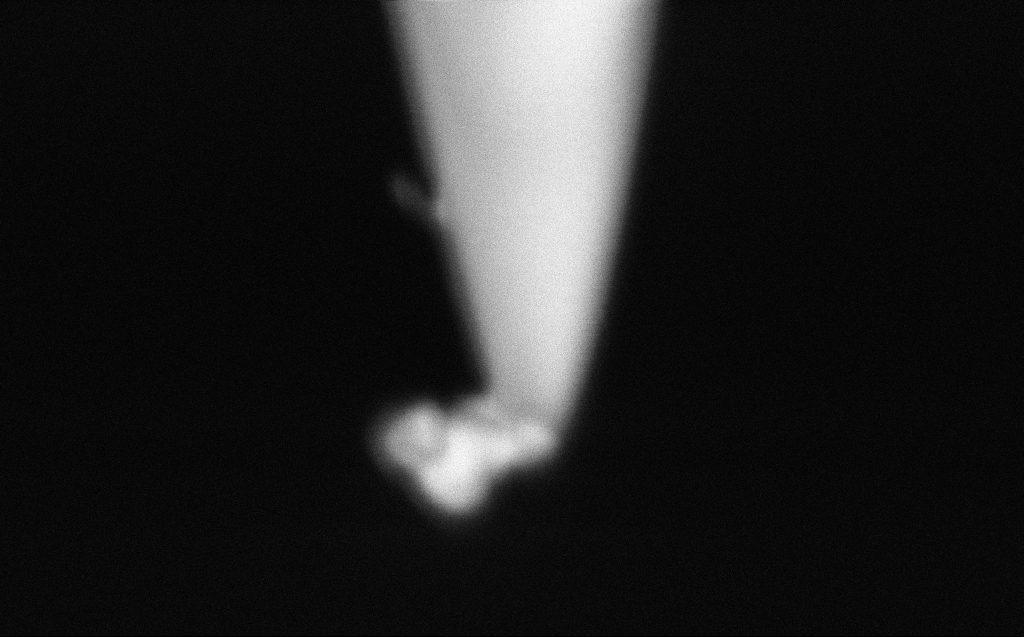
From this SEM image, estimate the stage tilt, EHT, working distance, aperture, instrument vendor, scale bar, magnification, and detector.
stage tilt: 45.1°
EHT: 1 kV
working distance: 5 mm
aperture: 30 µm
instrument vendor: Zeiss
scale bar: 200 nm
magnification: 185.77 K X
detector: InLens